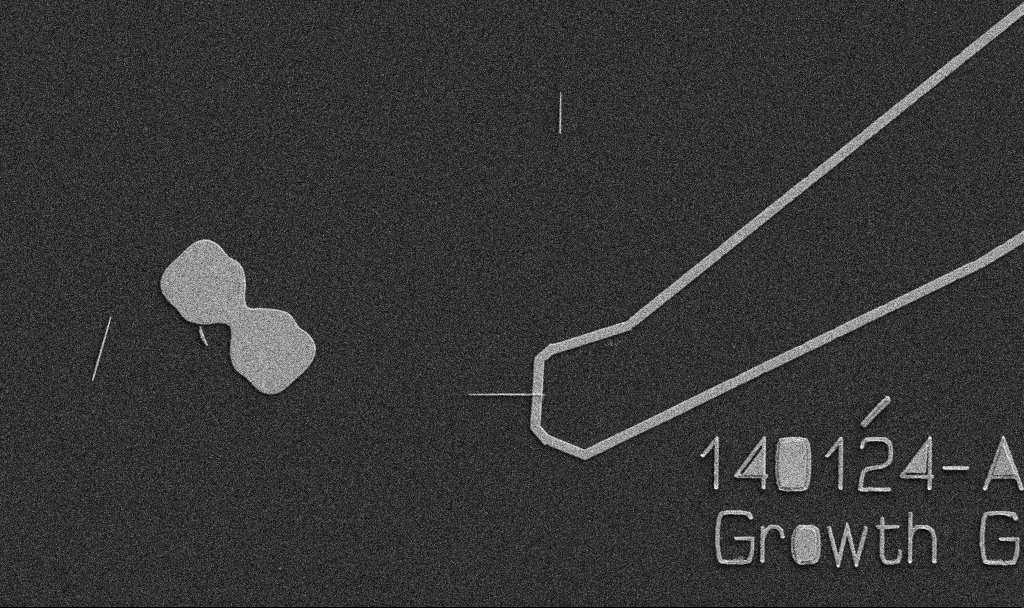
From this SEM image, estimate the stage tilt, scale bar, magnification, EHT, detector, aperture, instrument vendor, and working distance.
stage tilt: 0°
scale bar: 10000 nm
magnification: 5 K X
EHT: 5 kV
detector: SE2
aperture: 30 µm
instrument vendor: Zeiss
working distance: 10.7 mm